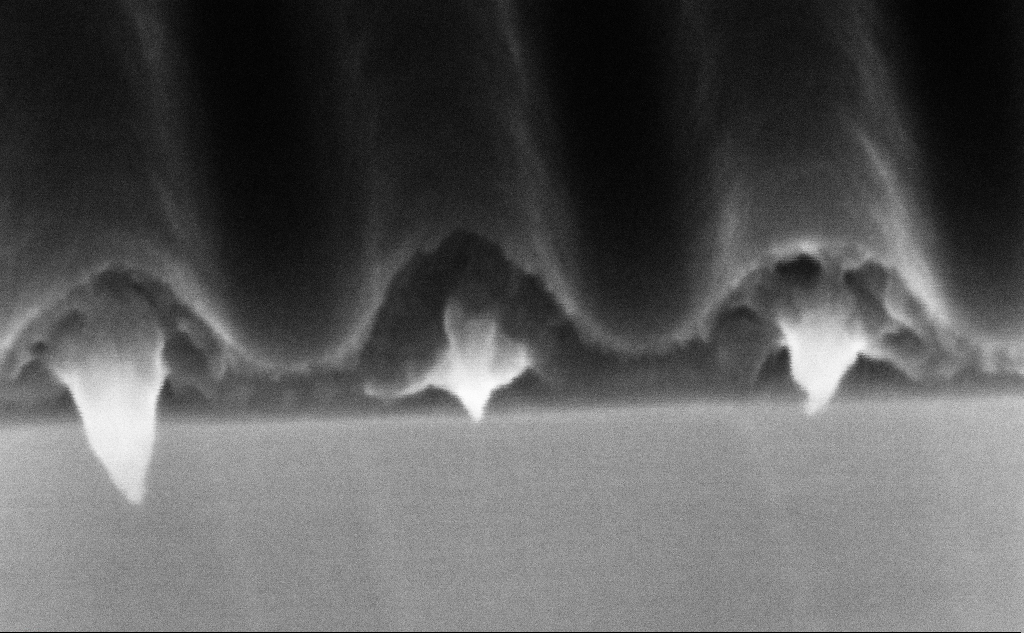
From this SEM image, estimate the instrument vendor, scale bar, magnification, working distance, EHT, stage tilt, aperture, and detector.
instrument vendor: Zeiss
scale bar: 100 nm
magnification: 433.13 K X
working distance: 7.4 mm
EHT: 5 kV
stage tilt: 45°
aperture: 30 µm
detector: InLens